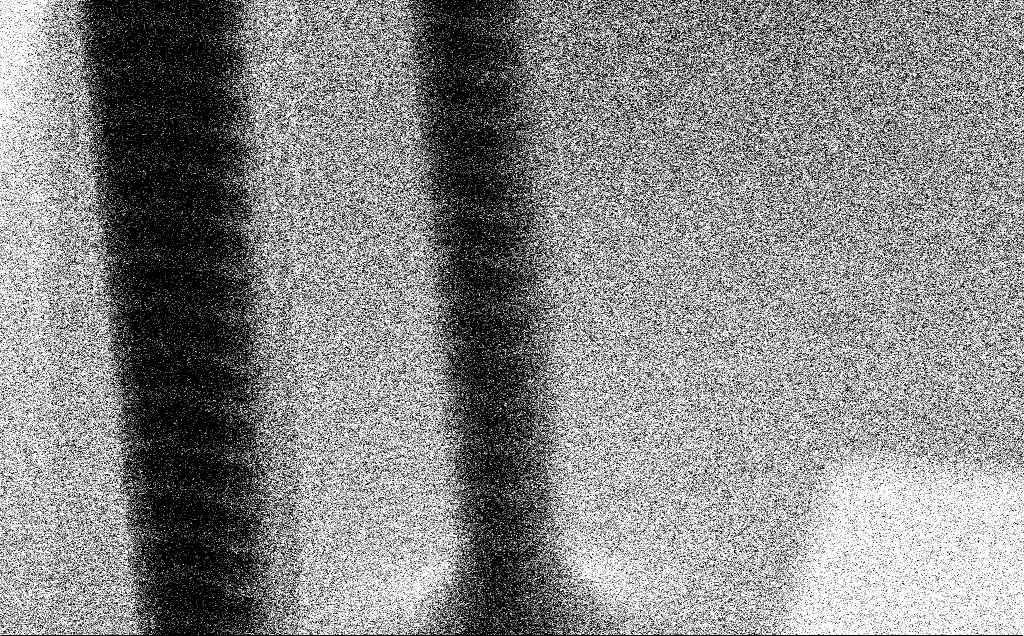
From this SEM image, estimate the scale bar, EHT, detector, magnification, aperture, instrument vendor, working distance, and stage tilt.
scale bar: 1000 nm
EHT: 3 kV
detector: SE2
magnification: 47.28 K X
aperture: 30 µm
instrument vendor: Zeiss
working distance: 5.3 mm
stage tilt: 60°